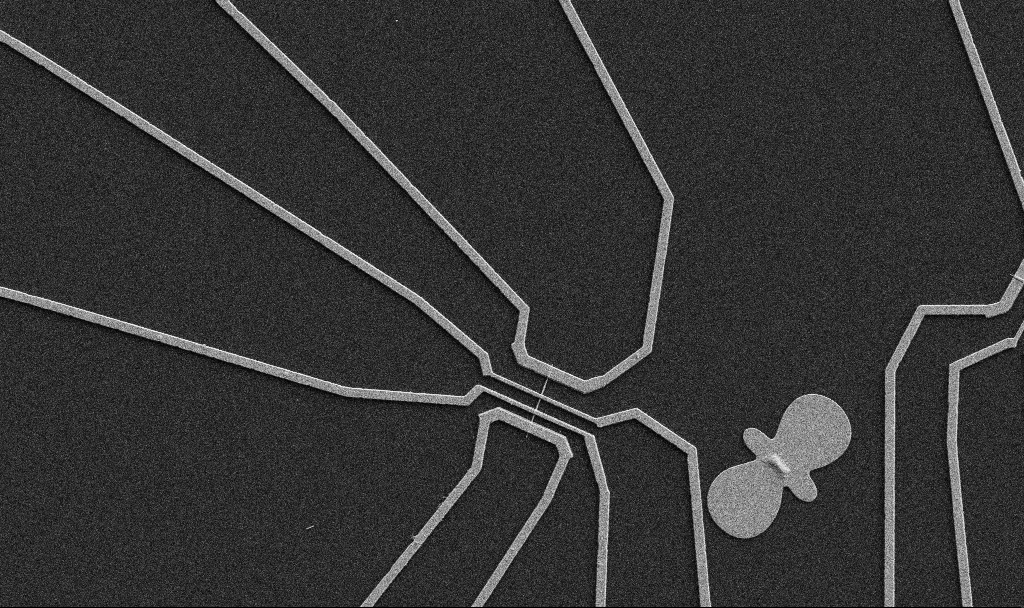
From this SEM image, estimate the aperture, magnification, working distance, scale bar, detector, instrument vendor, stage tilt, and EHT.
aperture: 30 µm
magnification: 5 K X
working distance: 10.7 mm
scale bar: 10000 nm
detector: SE2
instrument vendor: Zeiss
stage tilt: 0°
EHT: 5 kV